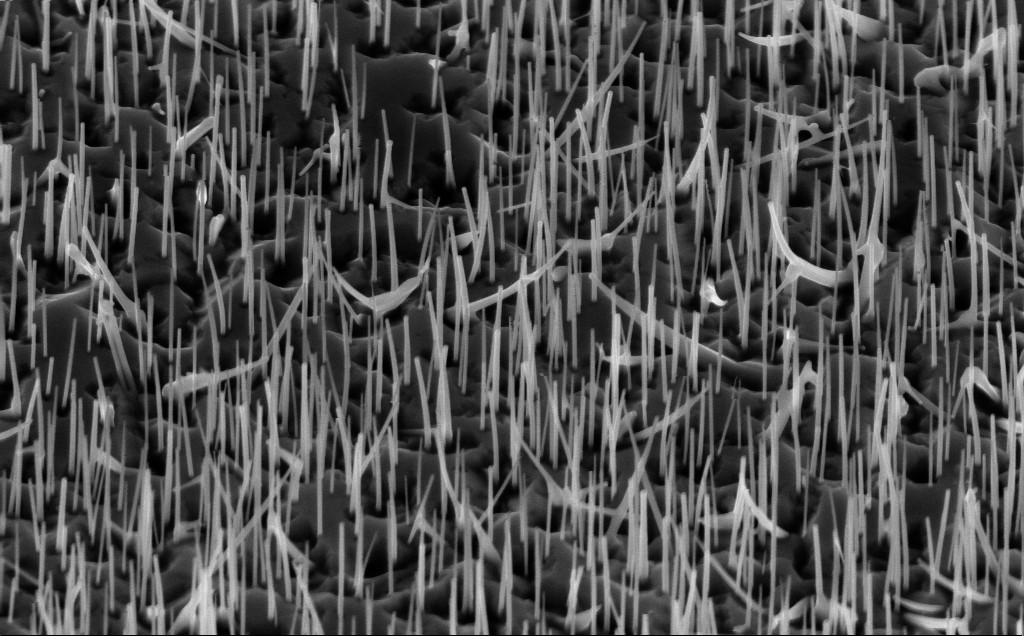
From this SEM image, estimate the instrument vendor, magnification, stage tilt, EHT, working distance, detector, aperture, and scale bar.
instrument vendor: Zeiss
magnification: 36.17 K X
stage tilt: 45°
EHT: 10 kV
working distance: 5 mm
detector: InLens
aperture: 30 µm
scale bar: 1000 nm